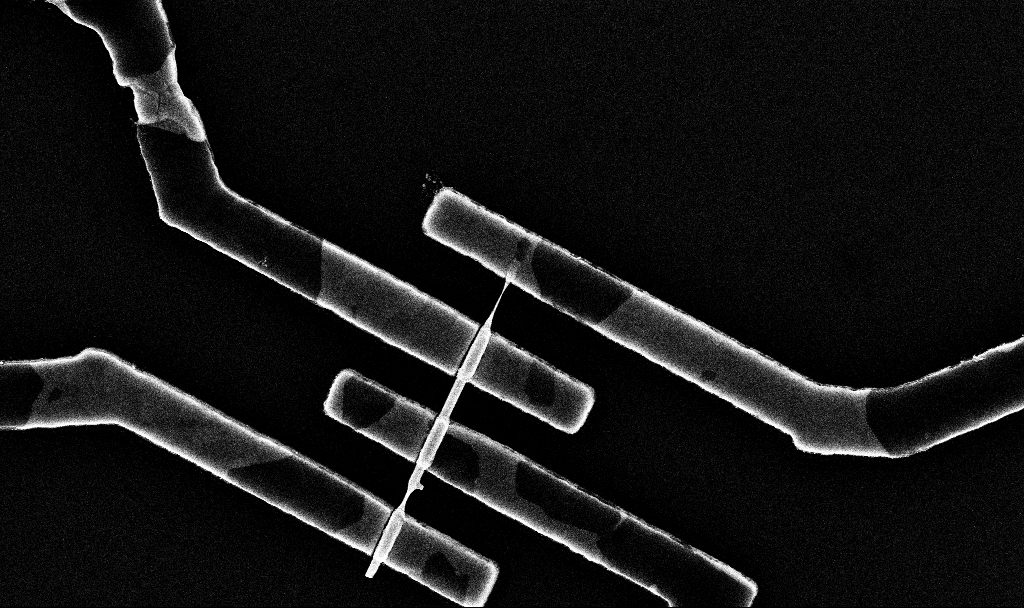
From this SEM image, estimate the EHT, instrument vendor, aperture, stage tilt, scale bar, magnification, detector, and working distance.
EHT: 10 kV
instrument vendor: Zeiss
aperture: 30 µm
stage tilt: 0°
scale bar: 2000 nm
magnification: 34.13 K X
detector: InLens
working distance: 6.8 mm